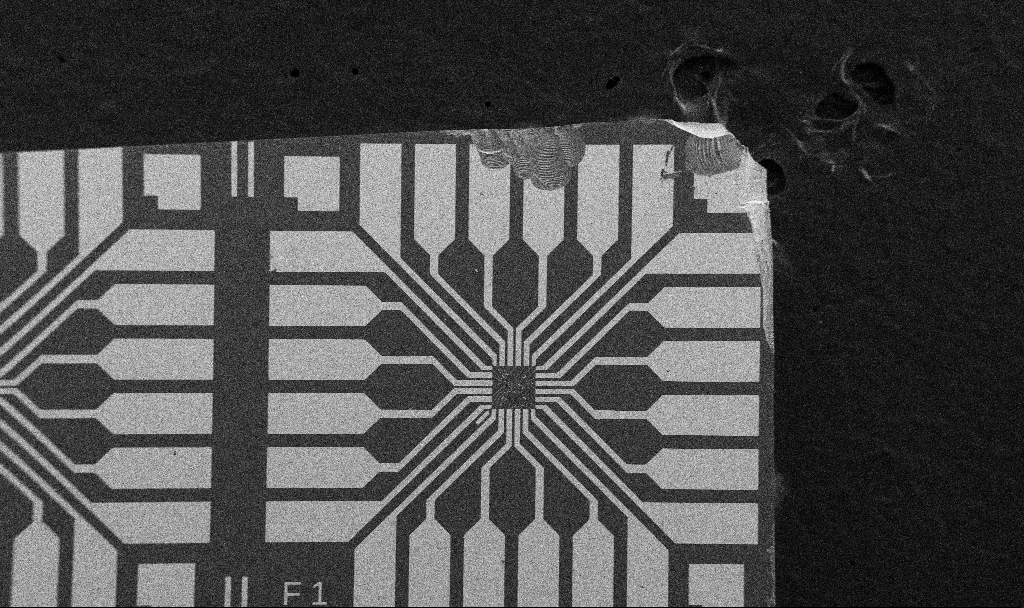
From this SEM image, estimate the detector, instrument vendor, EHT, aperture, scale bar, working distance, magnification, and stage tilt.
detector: SE2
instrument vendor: Zeiss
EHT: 5 kV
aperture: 30 µm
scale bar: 200000 nm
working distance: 10.7 mm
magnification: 0.1 K X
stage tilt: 0°